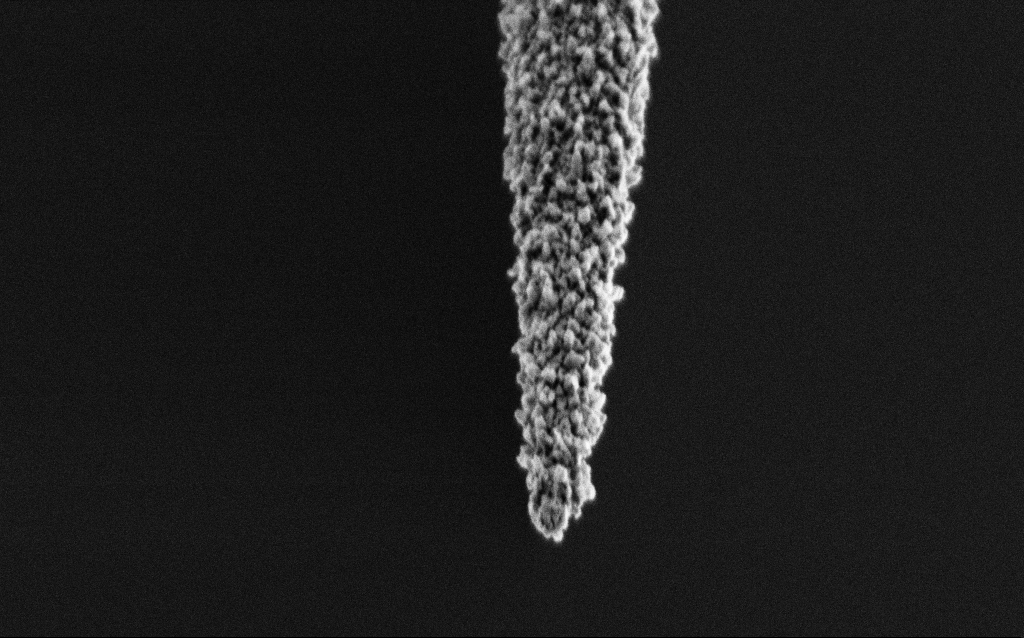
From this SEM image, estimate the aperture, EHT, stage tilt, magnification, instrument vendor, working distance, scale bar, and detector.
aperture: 30 µm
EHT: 2 kV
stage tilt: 44.9°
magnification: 40 K X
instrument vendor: Zeiss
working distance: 6.5 mm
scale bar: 1000 nm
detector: SE2